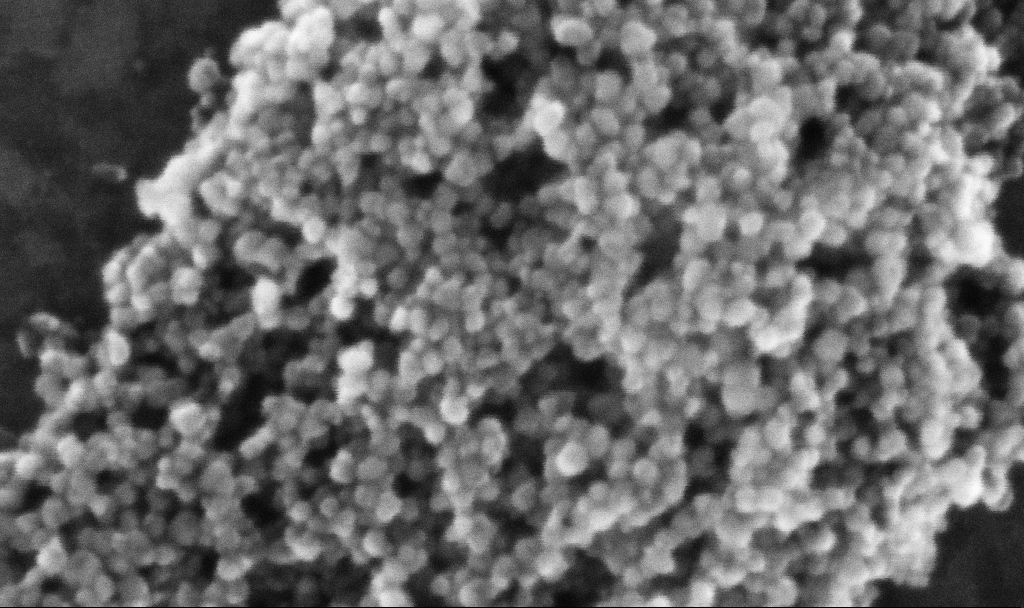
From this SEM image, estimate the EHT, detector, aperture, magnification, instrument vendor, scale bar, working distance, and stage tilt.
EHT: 10 kV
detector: InLens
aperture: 30 µm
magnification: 591.74 K X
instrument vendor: Zeiss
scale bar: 100 nm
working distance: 5.3 mm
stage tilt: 0°